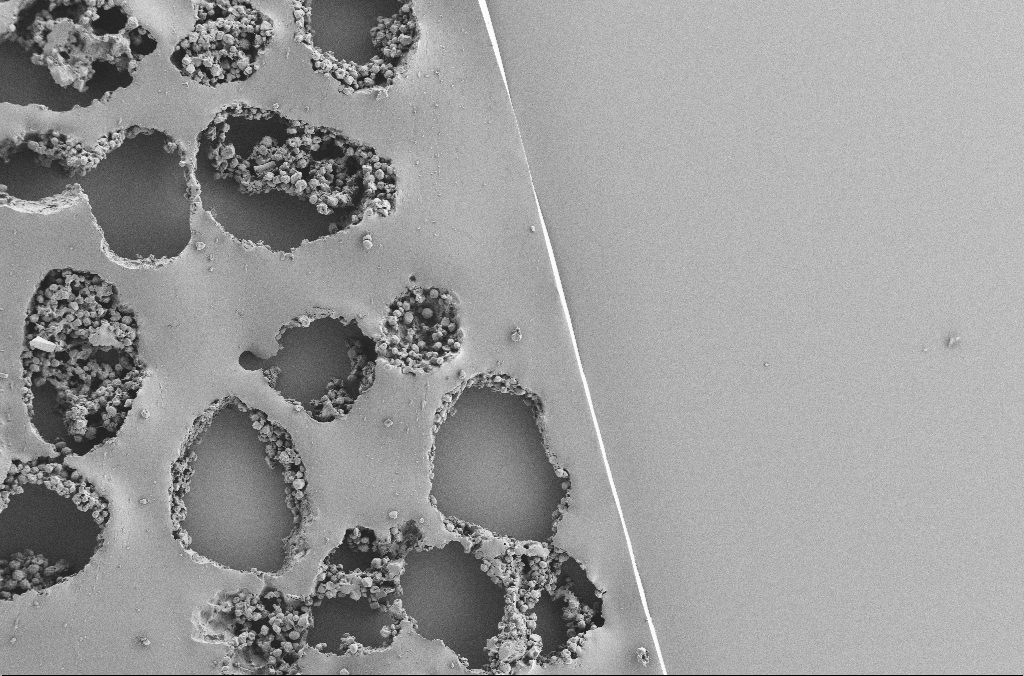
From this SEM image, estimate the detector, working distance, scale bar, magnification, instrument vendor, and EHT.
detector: SE2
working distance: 3.7 mm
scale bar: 100000 nm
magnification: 0.25 K X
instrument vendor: Zeiss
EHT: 2 kV